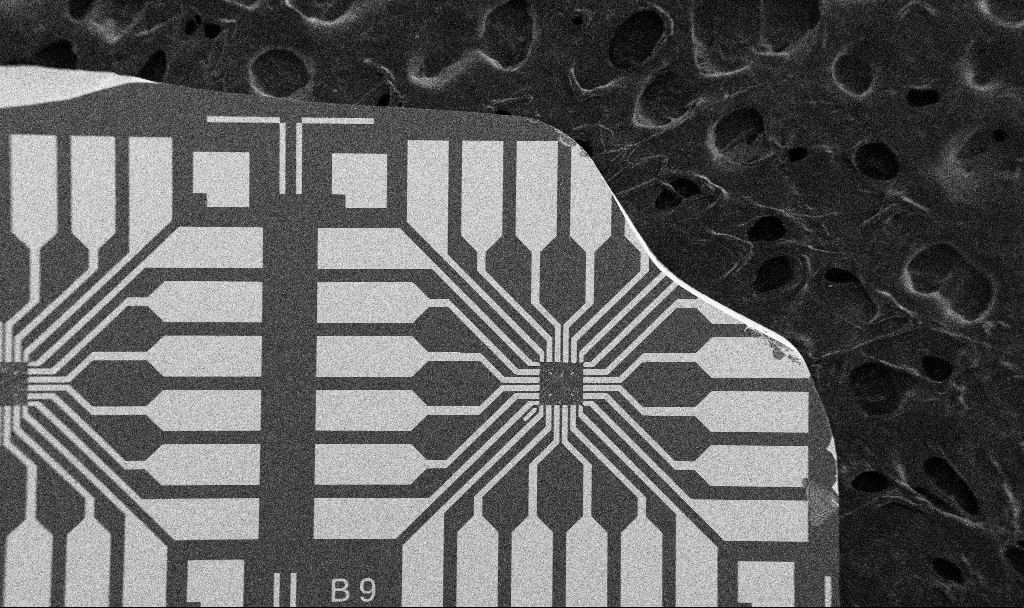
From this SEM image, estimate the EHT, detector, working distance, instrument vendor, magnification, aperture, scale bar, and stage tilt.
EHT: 5 kV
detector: SE2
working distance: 10.7 mm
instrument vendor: Zeiss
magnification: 0.1 K X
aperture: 30 µm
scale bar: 200000 nm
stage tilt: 0°